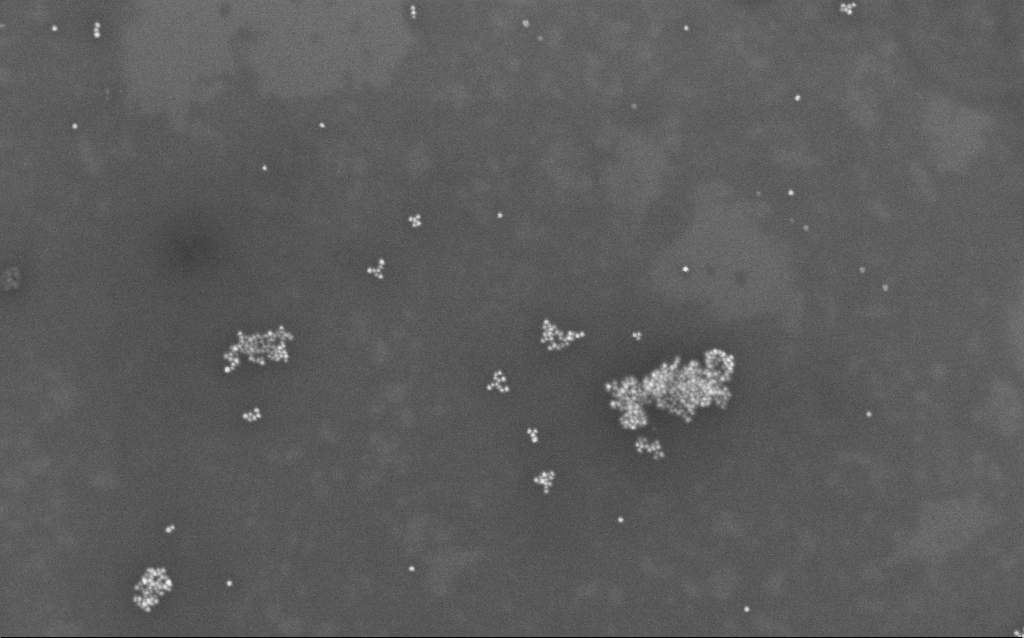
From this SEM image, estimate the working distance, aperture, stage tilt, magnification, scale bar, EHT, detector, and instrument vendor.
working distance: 7 mm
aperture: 30 µm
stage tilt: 0°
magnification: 100 K X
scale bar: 200 nm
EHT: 10 kV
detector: InLens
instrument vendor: Zeiss